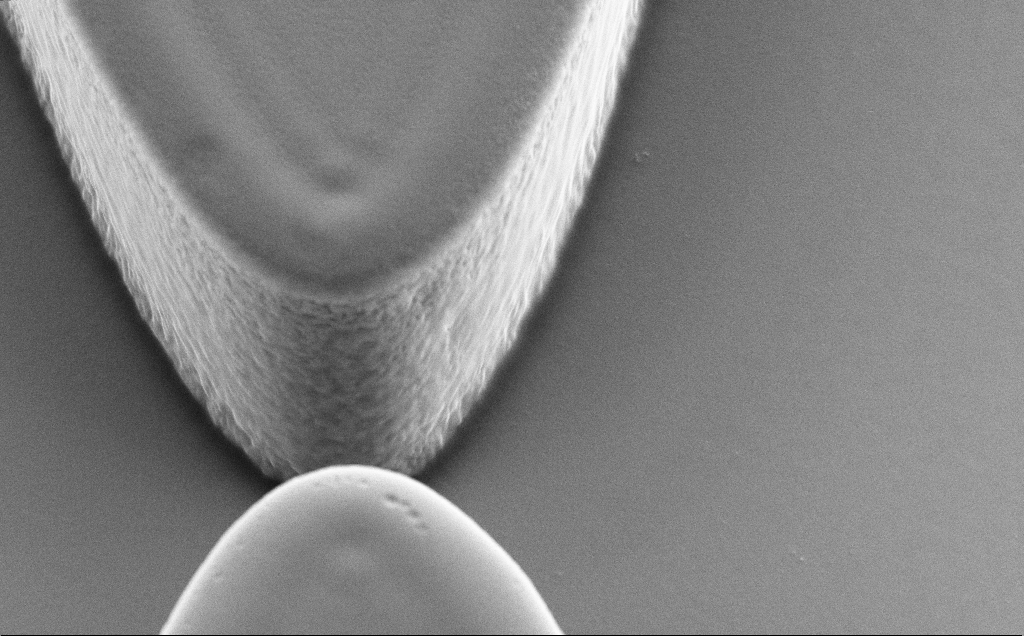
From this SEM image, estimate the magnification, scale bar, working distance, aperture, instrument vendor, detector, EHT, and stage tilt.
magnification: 16.39 K X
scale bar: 1000 nm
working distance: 11 mm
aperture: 30 µm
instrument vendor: Zeiss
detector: SE2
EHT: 5 kV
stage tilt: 30°